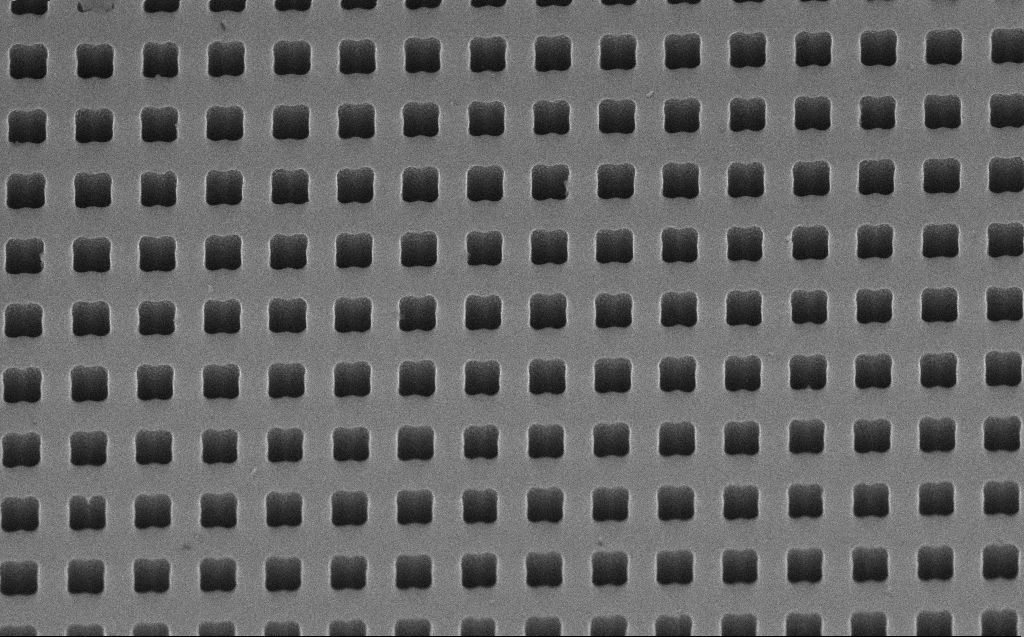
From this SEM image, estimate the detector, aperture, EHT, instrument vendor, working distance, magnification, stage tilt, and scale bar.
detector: InLens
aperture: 30 µm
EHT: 10 kV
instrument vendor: Zeiss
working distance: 7 mm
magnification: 48.33 K X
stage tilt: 45°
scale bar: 1000 nm